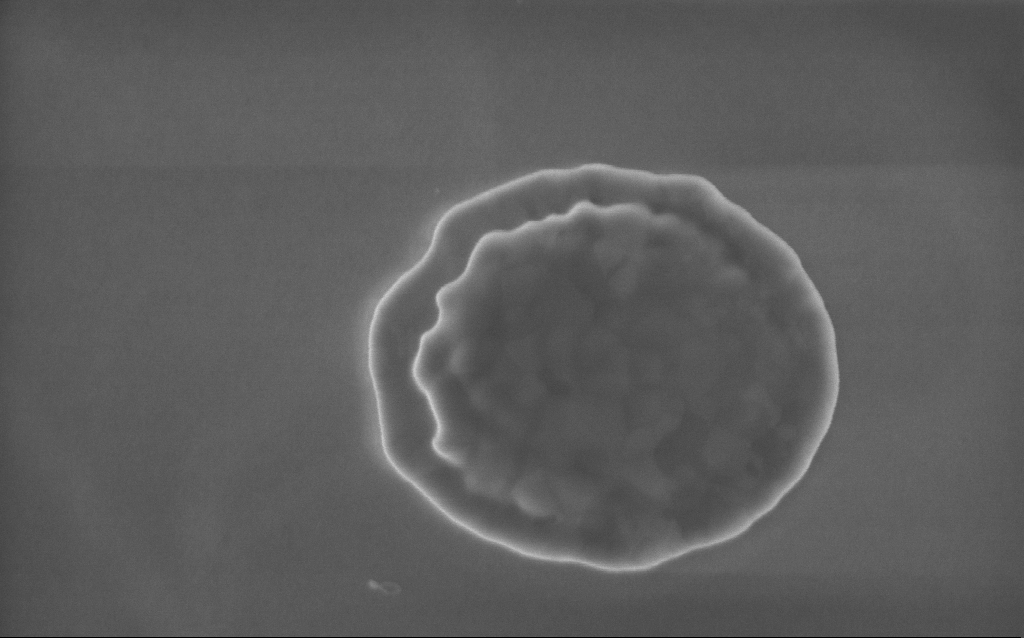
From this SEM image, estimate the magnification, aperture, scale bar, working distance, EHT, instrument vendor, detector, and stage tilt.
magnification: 89 K X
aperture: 30 µm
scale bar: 200 nm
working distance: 4 mm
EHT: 5 kV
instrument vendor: Zeiss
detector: InLens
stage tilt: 0°